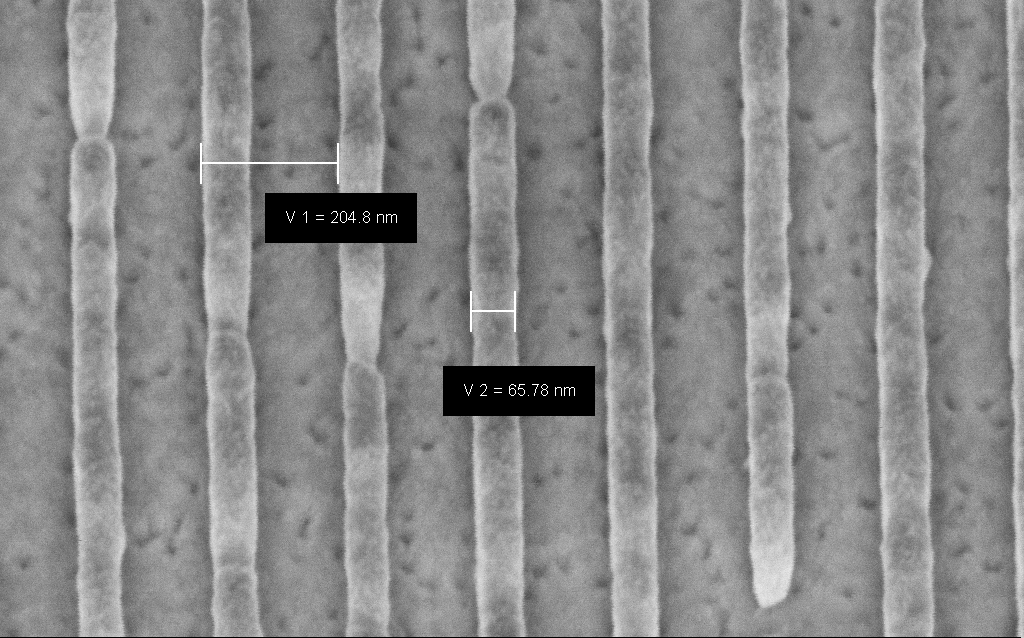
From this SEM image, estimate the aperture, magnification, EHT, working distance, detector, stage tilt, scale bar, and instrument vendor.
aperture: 30 µm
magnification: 245.62 K X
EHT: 3 kV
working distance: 6.6 mm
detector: InLens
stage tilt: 45°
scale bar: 200 nm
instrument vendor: Zeiss